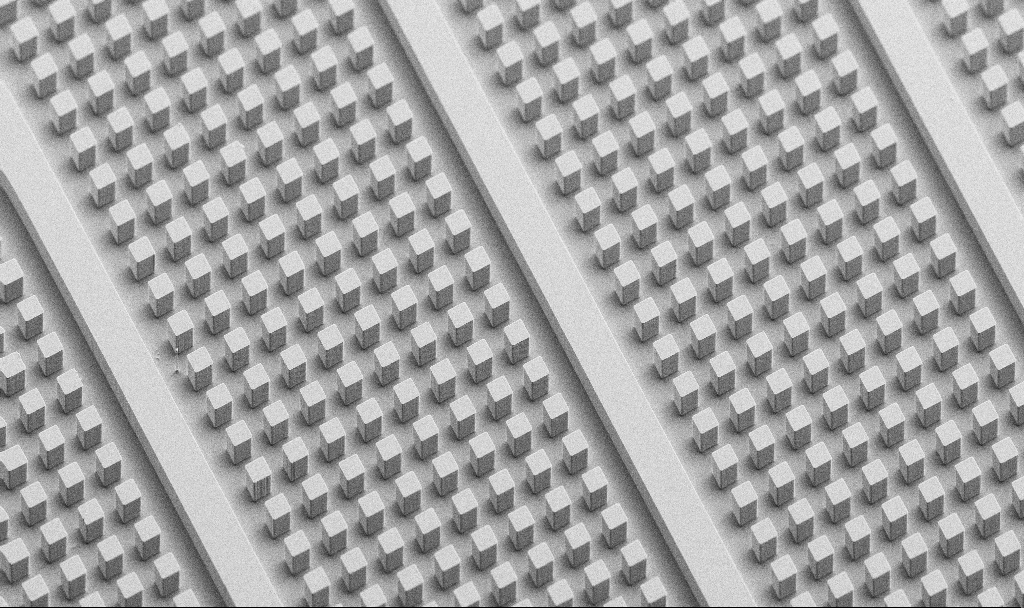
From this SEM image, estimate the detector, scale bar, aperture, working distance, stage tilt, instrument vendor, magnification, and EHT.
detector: SE2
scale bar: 20000 nm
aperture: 30 µm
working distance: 6.5 mm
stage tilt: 45°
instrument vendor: Zeiss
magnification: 0.772 K X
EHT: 5 kV